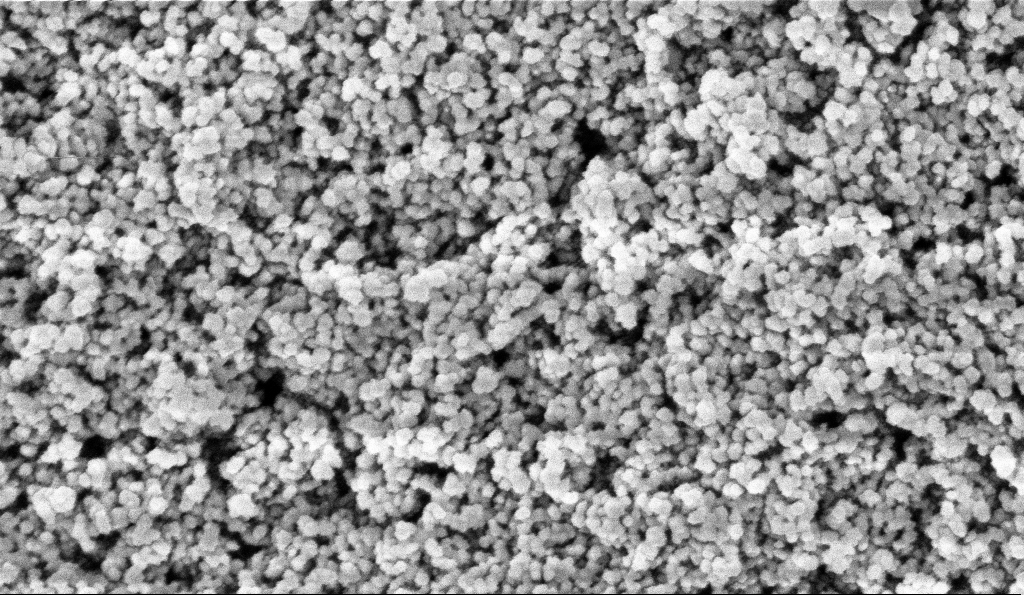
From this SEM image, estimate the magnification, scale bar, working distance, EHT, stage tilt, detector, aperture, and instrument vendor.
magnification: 135 K X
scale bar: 100 nm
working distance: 6 mm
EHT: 5 kV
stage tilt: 0°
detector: InLens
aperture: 30 µm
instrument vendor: Zeiss